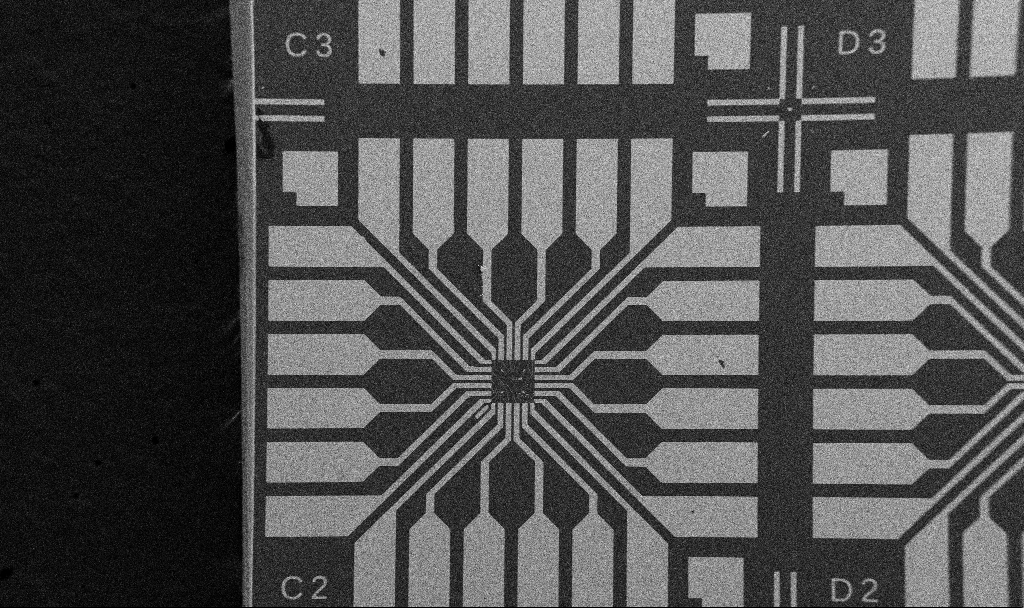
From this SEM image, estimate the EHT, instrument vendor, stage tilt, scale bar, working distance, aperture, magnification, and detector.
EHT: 5 kV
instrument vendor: Zeiss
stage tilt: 0°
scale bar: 200000 nm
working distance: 10.7 mm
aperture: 30 µm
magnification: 0.1 K X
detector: SE2